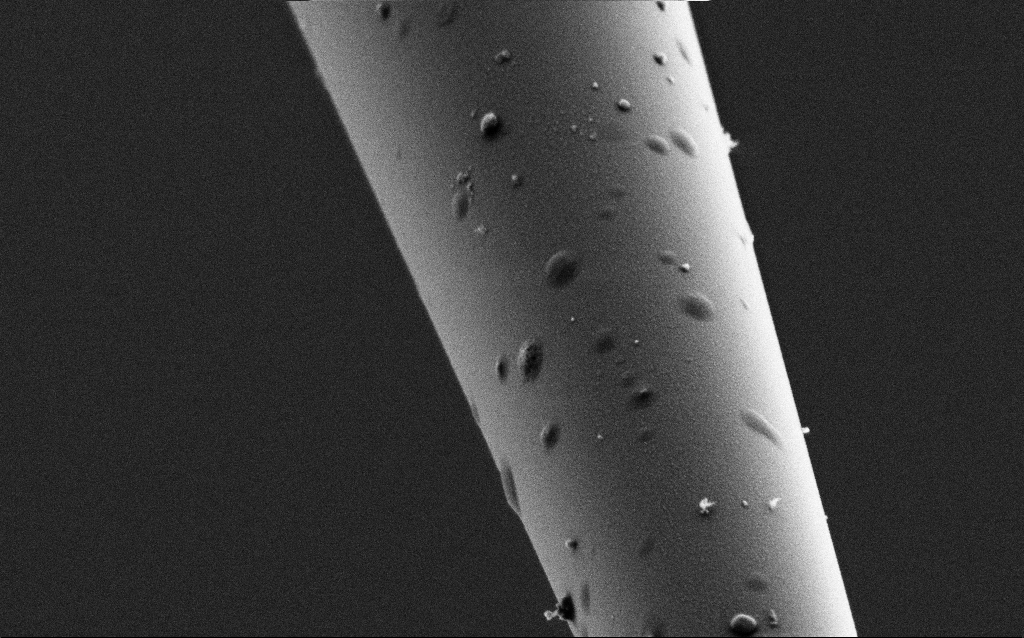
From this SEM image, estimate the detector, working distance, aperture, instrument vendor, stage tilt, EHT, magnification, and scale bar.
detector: SE2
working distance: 7.8 mm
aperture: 30 µm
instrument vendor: Zeiss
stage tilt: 45°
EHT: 2 kV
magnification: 25 K X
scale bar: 2000 nm